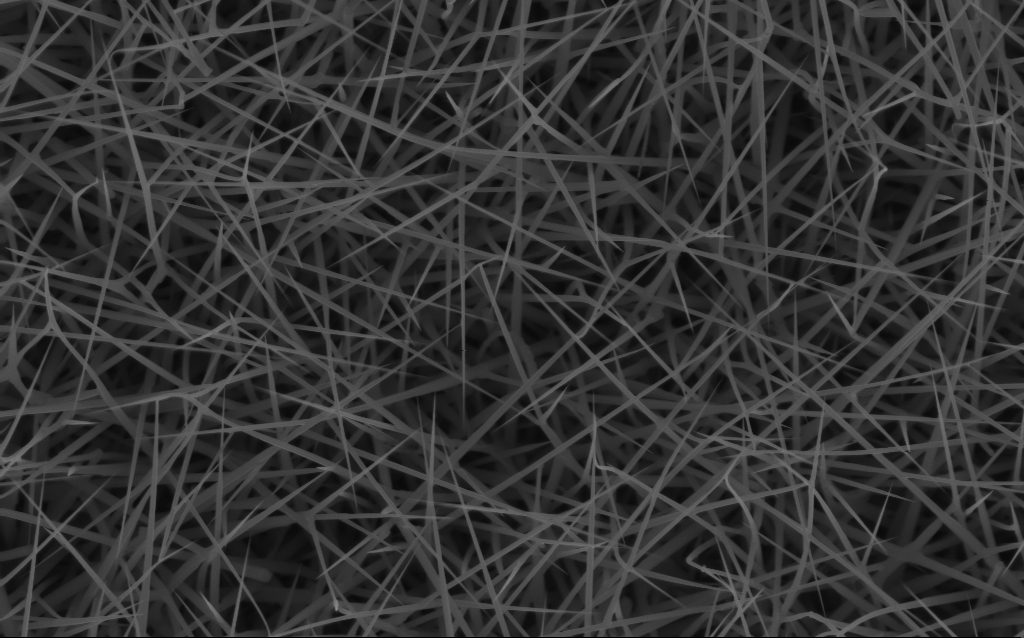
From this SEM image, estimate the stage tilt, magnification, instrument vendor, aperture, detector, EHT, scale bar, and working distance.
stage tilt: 0°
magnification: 20 K X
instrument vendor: Zeiss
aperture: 30 µm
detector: InLens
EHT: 10 kV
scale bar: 2000 nm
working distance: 6 mm